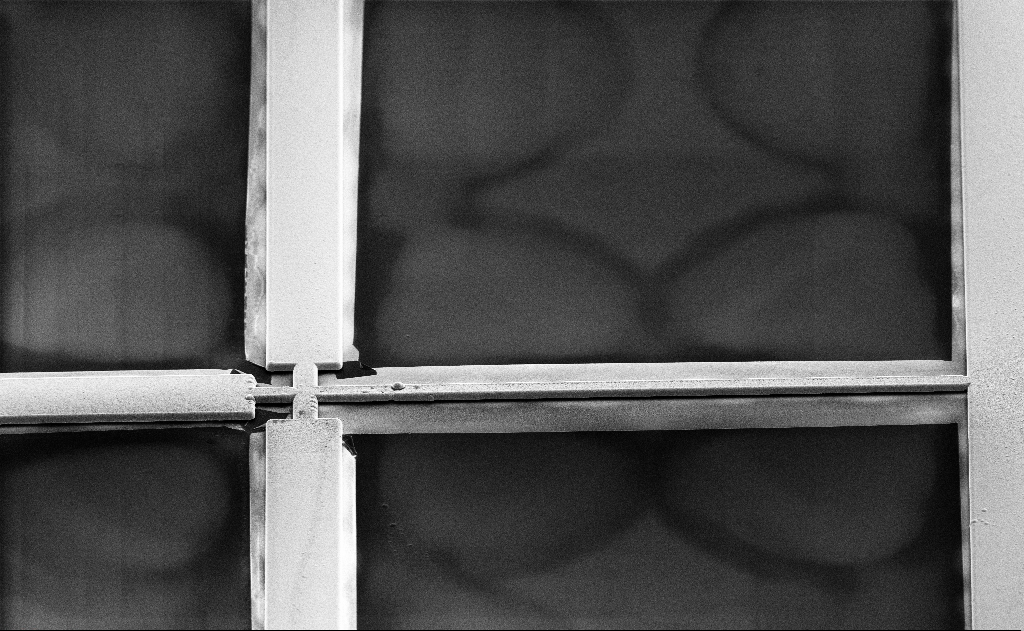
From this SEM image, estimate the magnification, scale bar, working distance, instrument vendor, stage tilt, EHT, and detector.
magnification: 0.476 K X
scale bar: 100000 nm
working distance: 13 mm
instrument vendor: Zeiss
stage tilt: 60°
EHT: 10 kV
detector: SE2